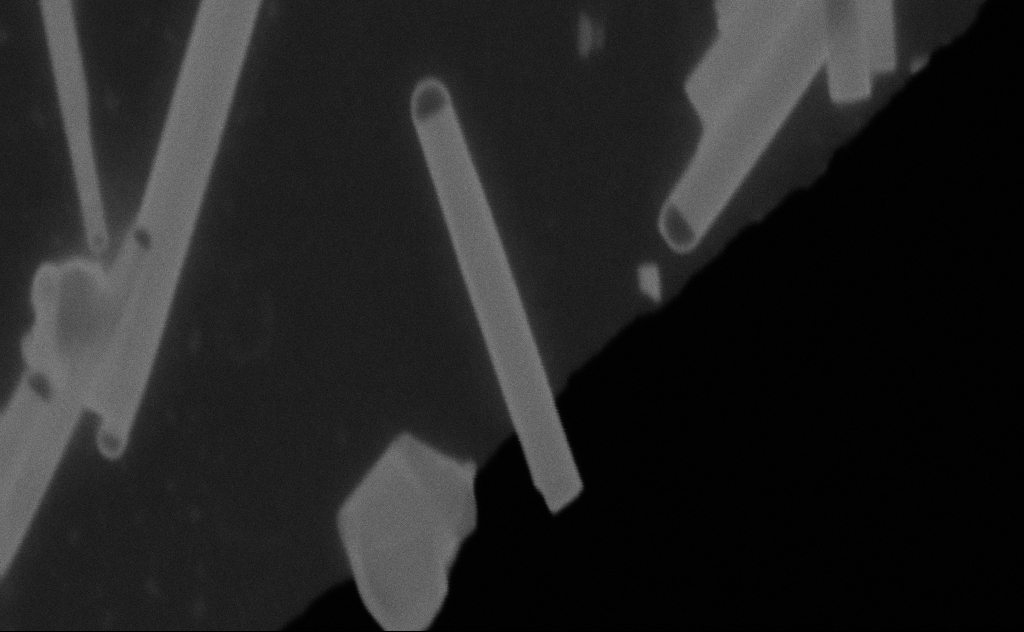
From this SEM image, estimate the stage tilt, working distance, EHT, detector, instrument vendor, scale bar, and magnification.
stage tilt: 0°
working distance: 9 mm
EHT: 20 kV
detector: SE2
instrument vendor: Zeiss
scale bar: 200 nm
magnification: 241.47 K X